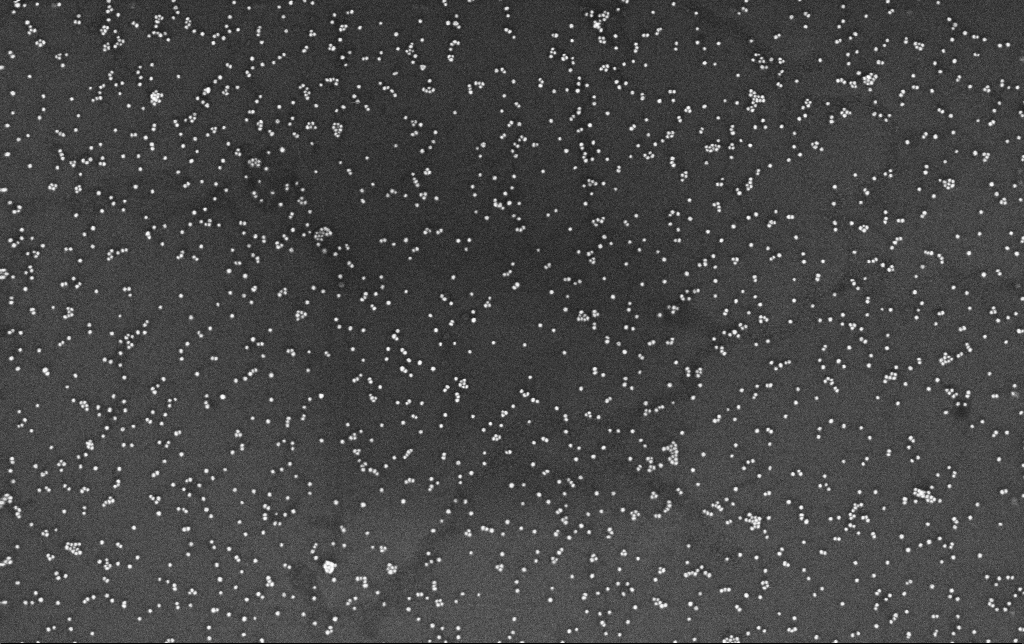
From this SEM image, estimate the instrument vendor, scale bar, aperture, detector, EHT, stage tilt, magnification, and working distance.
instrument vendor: Zeiss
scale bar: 200 nm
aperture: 30 µm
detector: InLens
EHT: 10 kV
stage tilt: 0°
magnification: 100 K X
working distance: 3.4 mm